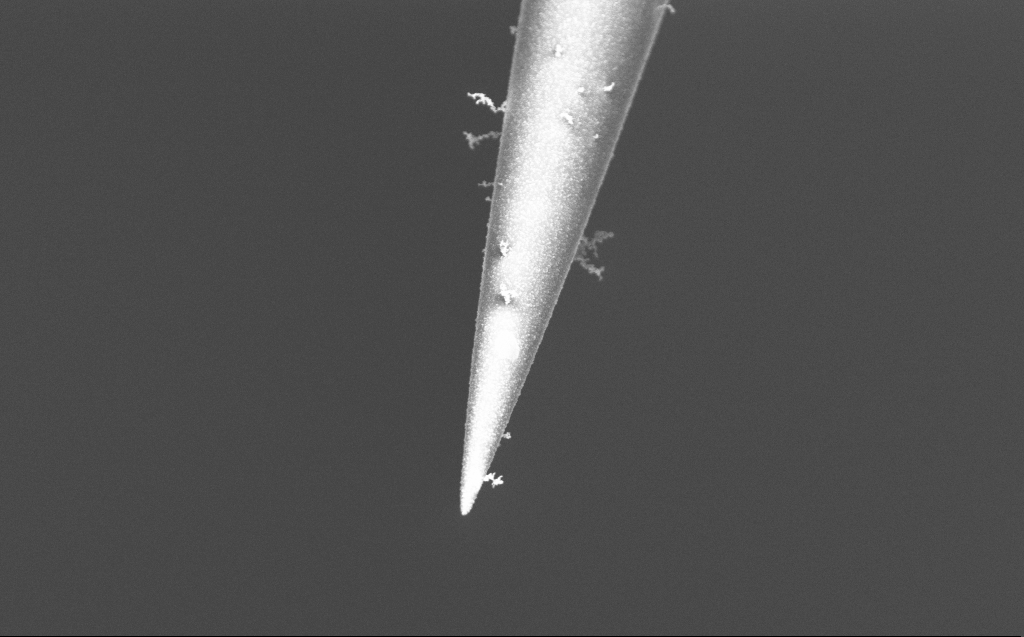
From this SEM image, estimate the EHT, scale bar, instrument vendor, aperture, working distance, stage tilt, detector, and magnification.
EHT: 2 kV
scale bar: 2000 nm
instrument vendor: Zeiss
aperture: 30 µm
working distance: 6 mm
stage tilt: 45°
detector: InLens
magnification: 20 K X